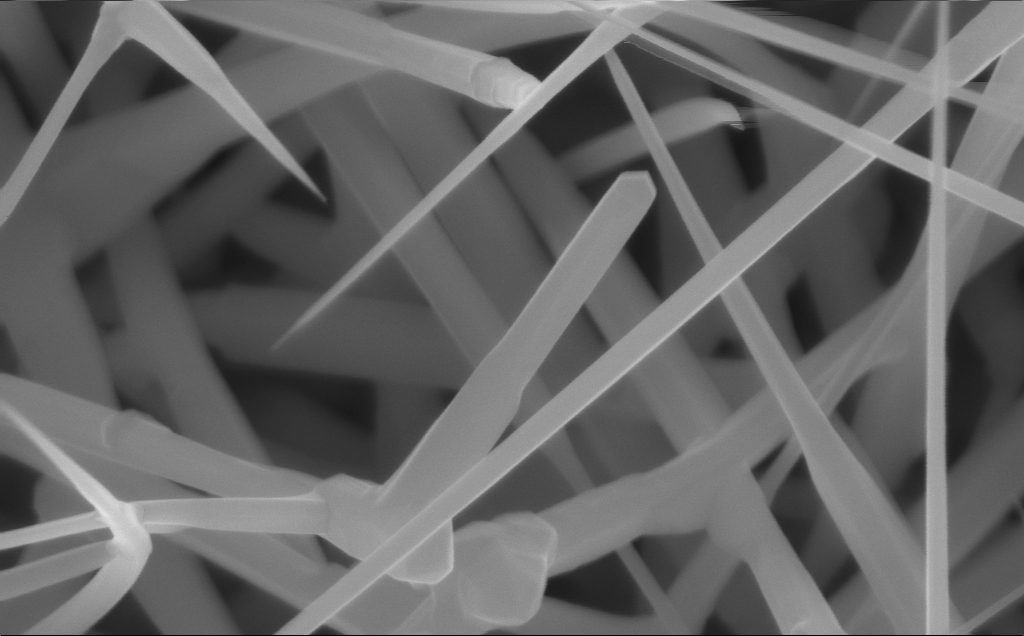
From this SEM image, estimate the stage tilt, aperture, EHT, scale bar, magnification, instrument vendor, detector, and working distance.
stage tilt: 0°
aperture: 30 µm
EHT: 10 kV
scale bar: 200 nm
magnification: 135.95 K X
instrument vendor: Zeiss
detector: InLens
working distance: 6 mm